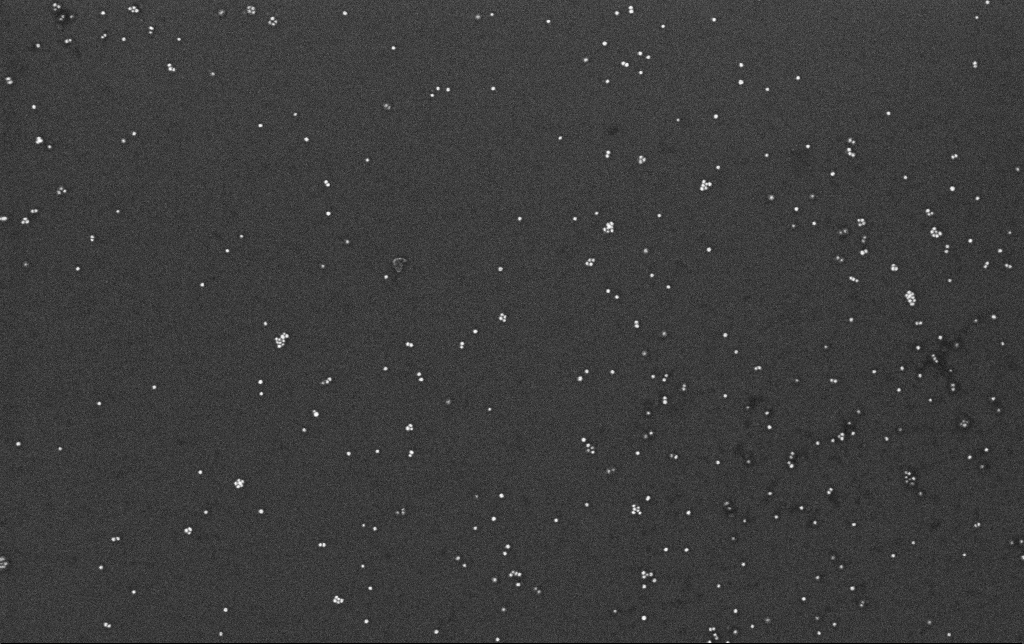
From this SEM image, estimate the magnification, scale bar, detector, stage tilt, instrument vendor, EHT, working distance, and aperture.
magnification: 100 K X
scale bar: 200 nm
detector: InLens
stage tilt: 0°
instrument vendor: Zeiss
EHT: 10 kV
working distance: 3.4 mm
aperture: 30 µm